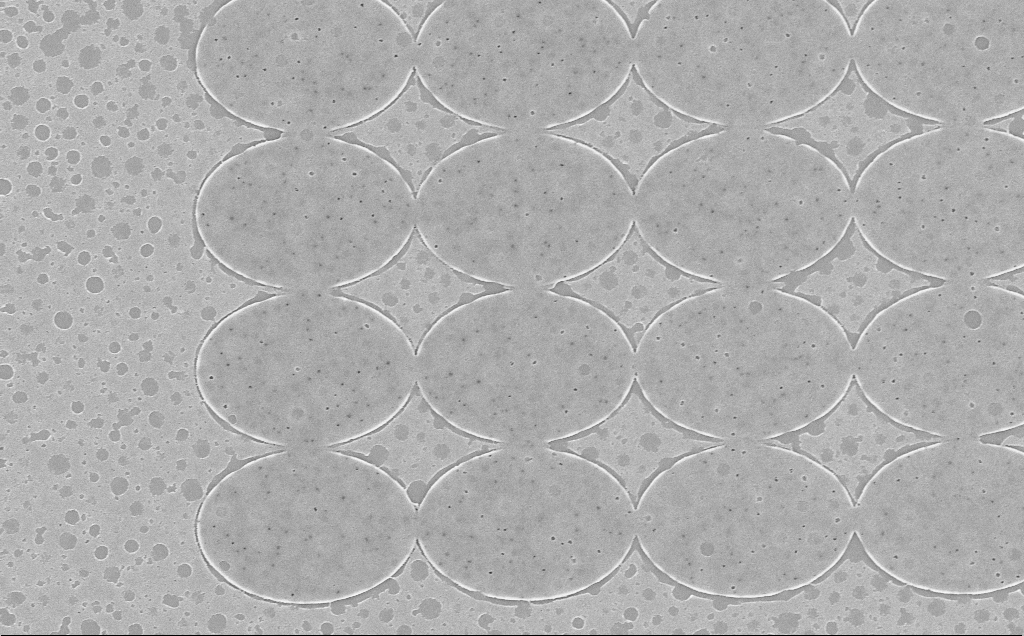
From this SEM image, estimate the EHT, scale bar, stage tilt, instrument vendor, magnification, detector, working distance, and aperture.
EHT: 5 kV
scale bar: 2000 nm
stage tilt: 0°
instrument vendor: Zeiss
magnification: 8.08 K X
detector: InLens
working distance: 6 mm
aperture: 30 µm